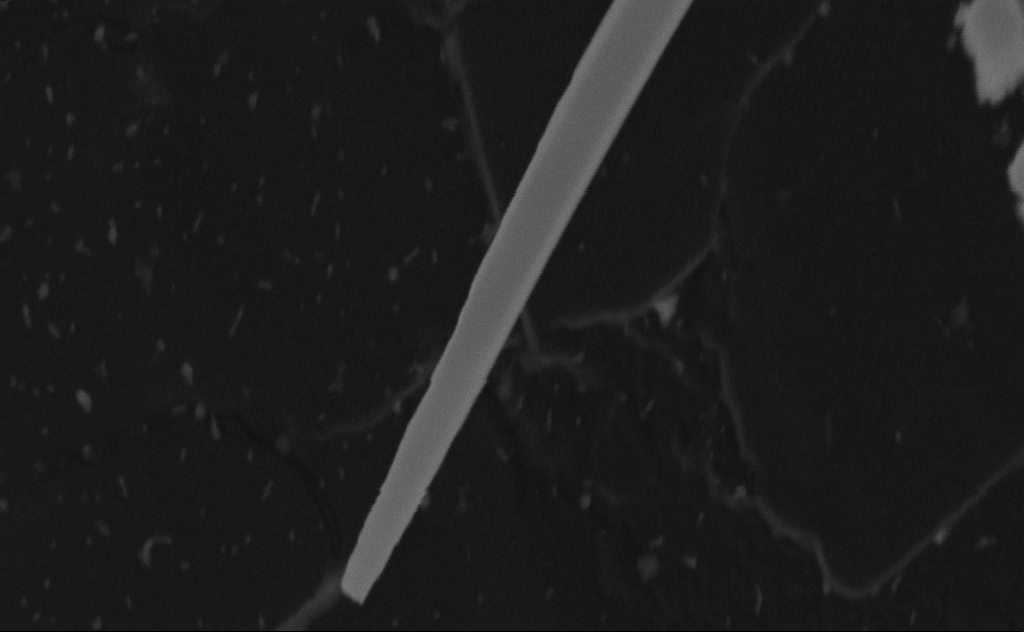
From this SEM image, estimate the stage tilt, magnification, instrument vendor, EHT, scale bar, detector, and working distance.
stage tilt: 0°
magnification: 192.47 K X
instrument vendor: Zeiss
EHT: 20 kV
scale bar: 200 nm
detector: SE2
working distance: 8 mm